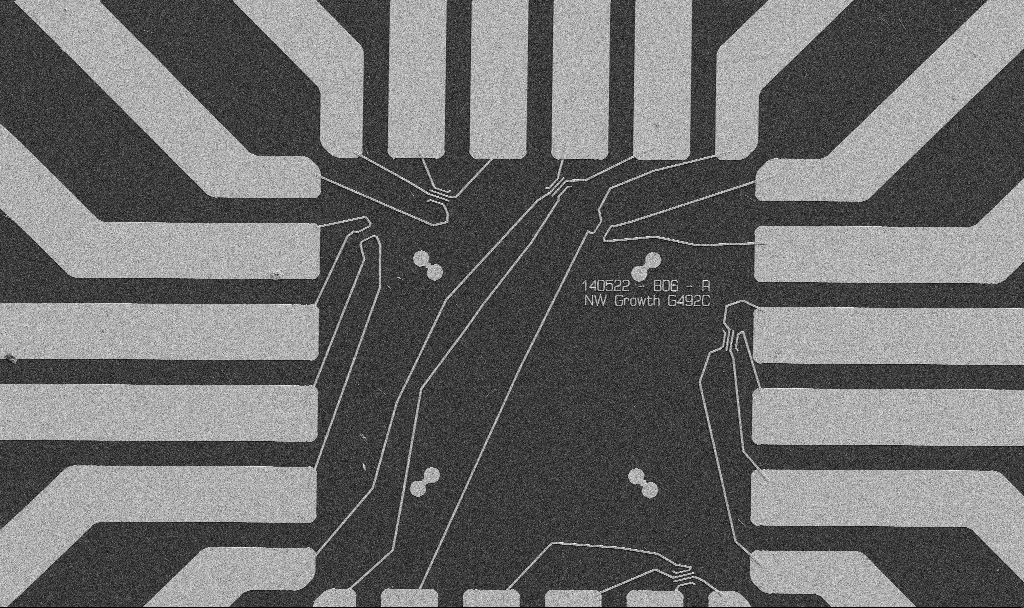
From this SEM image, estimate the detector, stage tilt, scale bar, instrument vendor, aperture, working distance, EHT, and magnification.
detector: SE2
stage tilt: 0°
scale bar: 20000 nm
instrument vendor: Zeiss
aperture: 30 µm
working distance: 10.7 mm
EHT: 5 kV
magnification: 1 K X